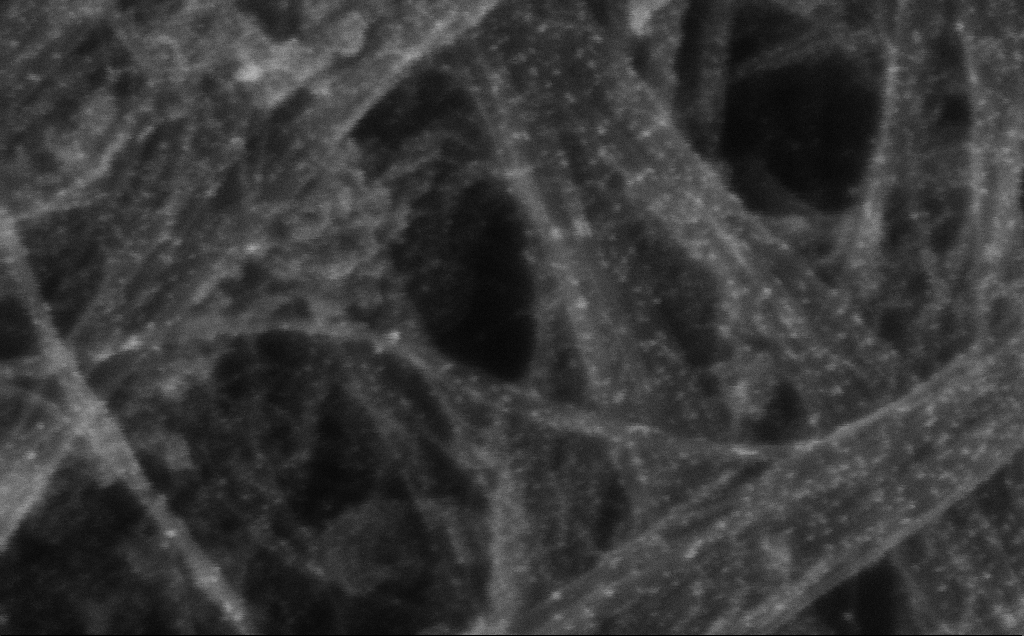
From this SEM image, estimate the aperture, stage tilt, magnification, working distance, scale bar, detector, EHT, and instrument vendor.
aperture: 30 µm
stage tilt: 0°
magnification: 484.67 K X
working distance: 3 mm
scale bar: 100 nm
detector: InLens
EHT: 10 kV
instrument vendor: Zeiss